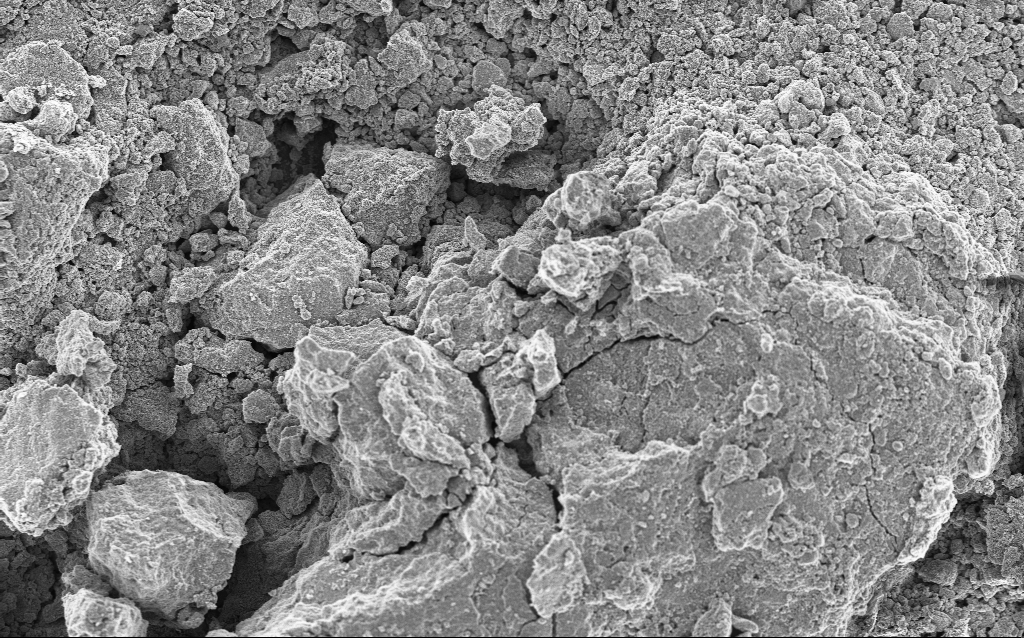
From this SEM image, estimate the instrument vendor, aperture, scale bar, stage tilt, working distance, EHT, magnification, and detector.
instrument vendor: Zeiss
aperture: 30 µm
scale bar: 10000 nm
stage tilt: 0°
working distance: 4.5 mm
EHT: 5 kV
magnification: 1.23 K X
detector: InLens